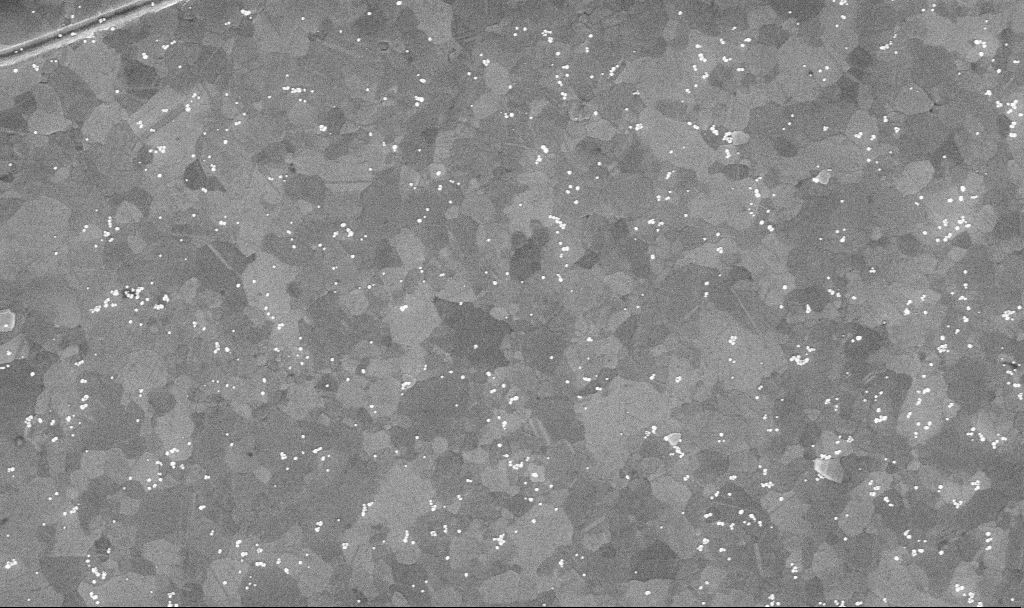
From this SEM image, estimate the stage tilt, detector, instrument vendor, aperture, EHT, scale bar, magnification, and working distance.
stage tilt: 0°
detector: InLens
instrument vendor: Zeiss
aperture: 30 µm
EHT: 10 kV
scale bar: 1000 nm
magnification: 50.84 K X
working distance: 3.4 mm